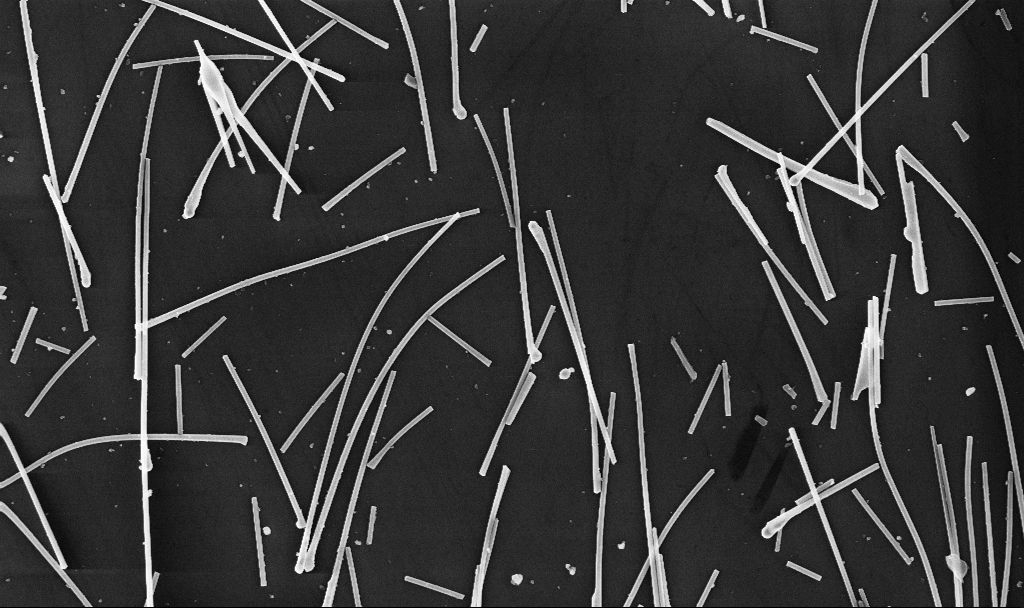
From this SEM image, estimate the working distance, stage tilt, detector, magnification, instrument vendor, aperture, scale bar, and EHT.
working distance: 6.7 mm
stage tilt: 0°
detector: InLens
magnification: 19.08 K X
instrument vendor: Zeiss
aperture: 30 µm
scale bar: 2000 nm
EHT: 10 kV